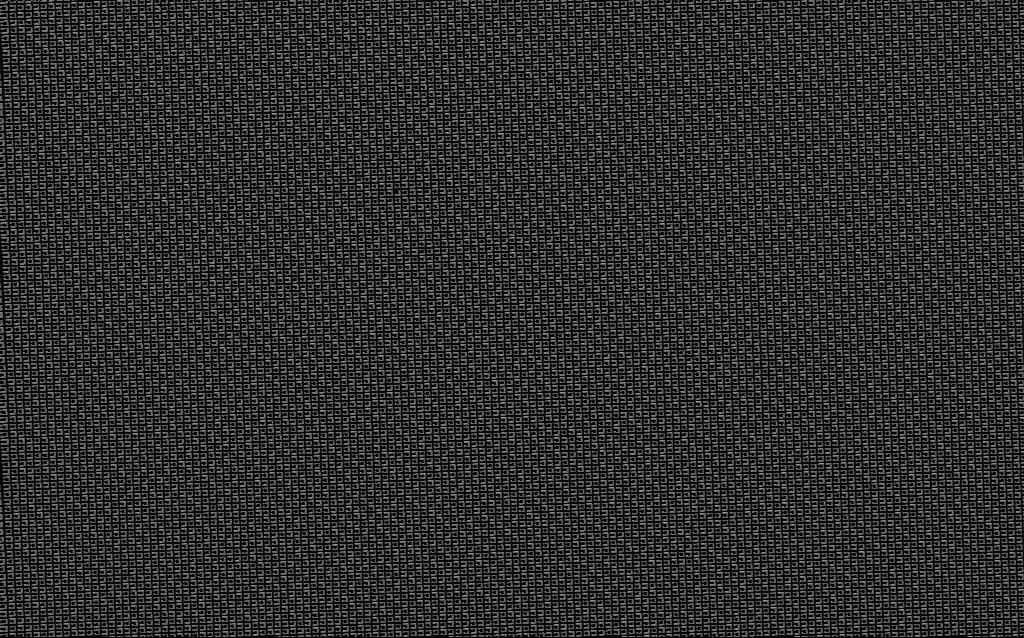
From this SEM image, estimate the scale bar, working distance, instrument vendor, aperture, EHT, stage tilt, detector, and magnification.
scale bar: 10000 nm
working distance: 6.8 mm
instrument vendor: Zeiss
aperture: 30 µm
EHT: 3 kV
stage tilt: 0°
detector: SE2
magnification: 6 K X